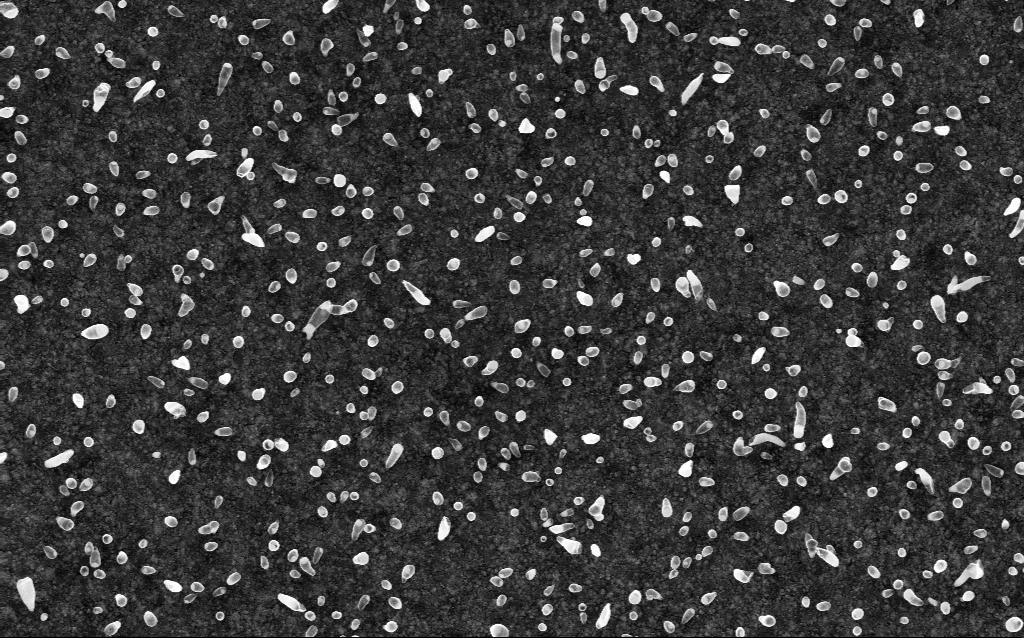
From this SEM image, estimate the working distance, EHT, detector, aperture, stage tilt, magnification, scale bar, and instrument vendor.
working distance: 1.9 mm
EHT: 5 kV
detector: InLens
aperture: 30 µm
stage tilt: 0°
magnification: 50 K X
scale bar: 1000 nm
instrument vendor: Zeiss